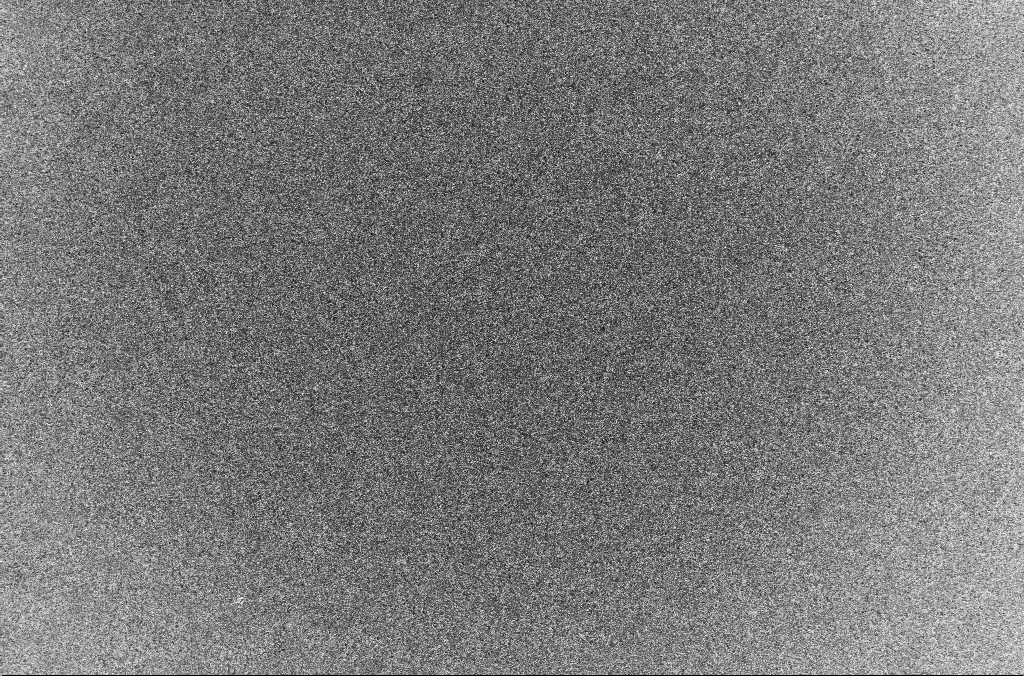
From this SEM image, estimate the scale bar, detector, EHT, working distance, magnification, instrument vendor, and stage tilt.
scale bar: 20000 nm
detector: InLens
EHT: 2 kV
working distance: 3 mm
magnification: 1 K X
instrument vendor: Zeiss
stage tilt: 0°